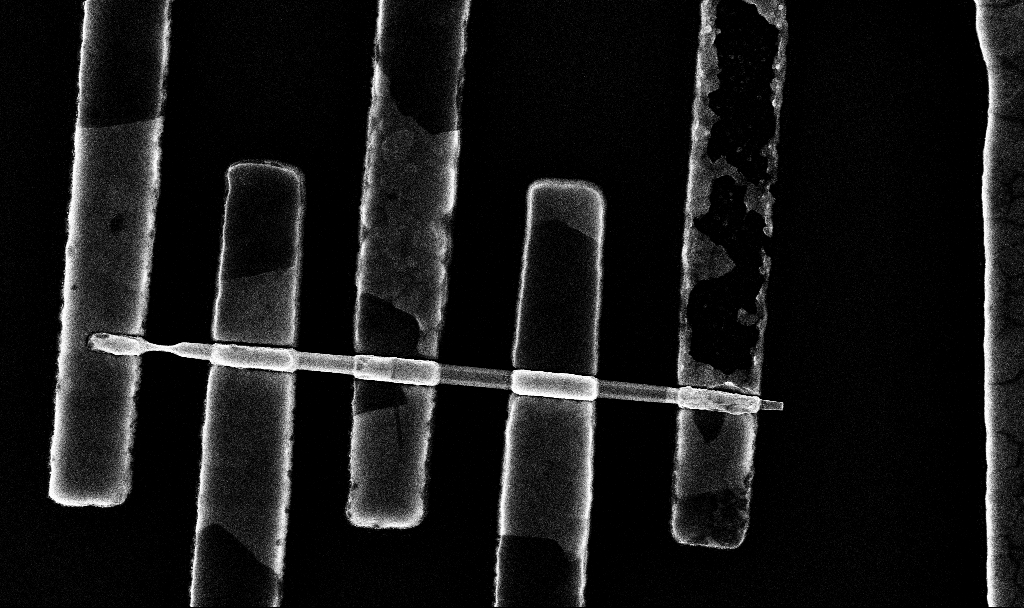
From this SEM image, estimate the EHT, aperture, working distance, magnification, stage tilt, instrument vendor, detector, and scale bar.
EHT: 10 kV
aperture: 30 µm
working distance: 6.8 mm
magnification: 50.67 K X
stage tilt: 0°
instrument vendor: Zeiss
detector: InLens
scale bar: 1000 nm